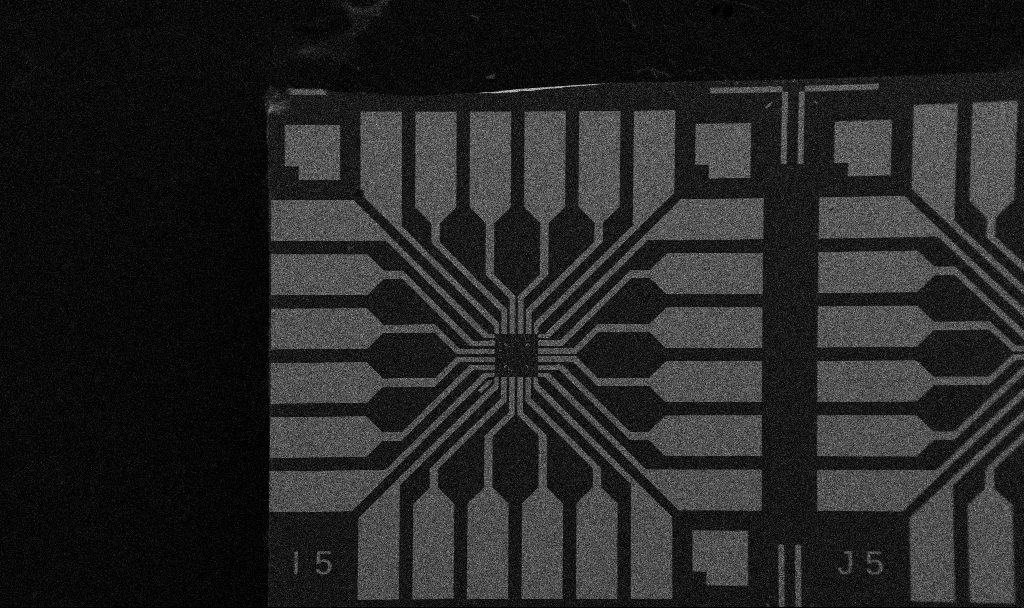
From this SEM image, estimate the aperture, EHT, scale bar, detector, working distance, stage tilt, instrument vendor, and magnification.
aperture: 30 µm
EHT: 5 kV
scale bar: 200000 nm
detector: SE2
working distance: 10.7 mm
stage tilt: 0°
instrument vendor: Zeiss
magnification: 0.1 K X